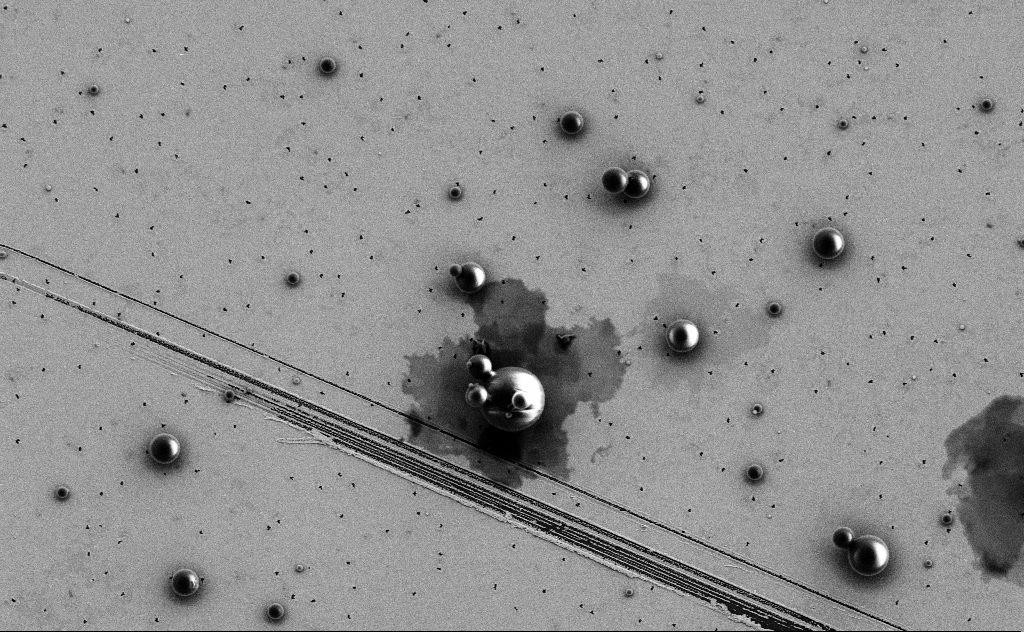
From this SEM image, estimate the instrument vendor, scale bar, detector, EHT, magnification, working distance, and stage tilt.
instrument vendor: Zeiss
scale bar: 10000 nm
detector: SE2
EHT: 3 kV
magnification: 6.73 K X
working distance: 11 mm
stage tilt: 0°